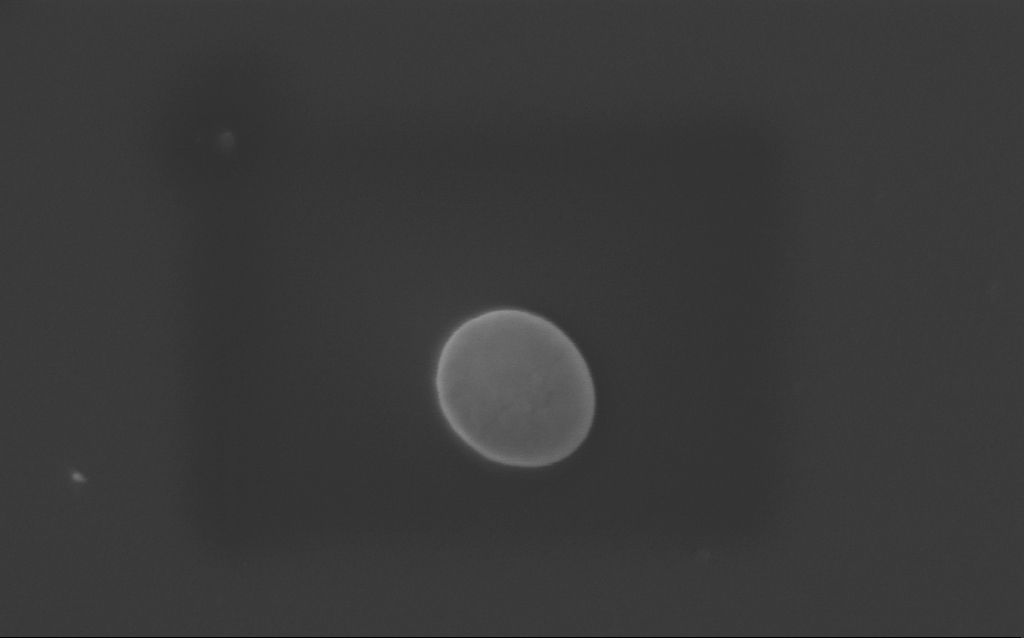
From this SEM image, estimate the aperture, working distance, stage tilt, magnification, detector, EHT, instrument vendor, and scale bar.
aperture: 30 µm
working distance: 3 mm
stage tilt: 0°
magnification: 132.71 K X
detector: InLens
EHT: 3 kV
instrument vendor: Zeiss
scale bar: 200 nm